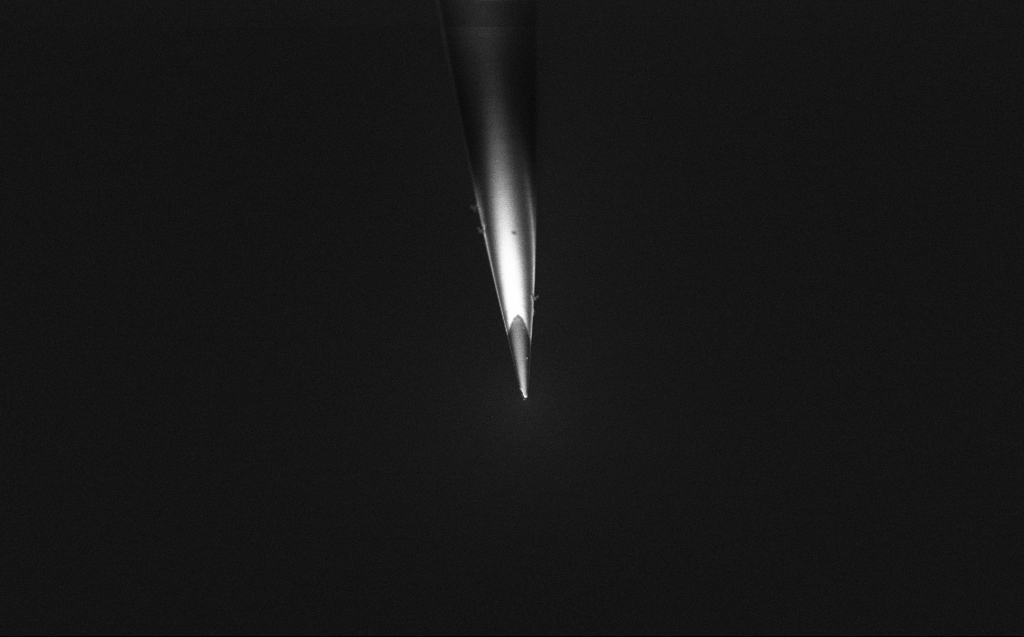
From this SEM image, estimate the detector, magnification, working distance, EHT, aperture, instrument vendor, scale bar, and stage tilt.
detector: InLens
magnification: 10 K X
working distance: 6 mm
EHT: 2 kV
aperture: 30 µm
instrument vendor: Zeiss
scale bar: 2000 nm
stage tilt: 45°